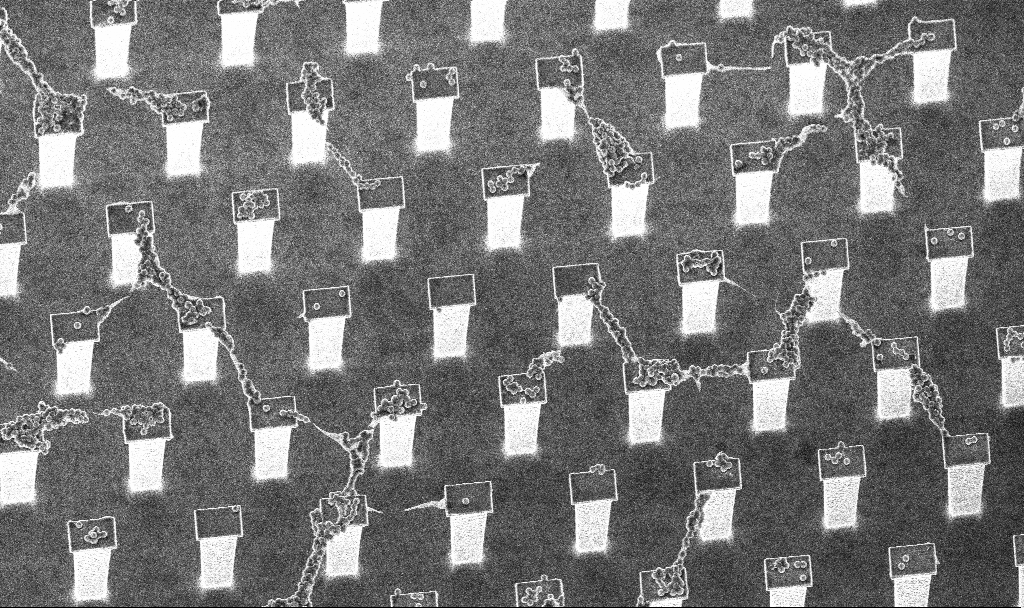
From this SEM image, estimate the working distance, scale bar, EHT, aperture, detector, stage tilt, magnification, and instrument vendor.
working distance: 3.2 mm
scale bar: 10000 nm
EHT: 5 kV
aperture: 30 µm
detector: InLens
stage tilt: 20°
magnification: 3.82 K X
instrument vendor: Zeiss